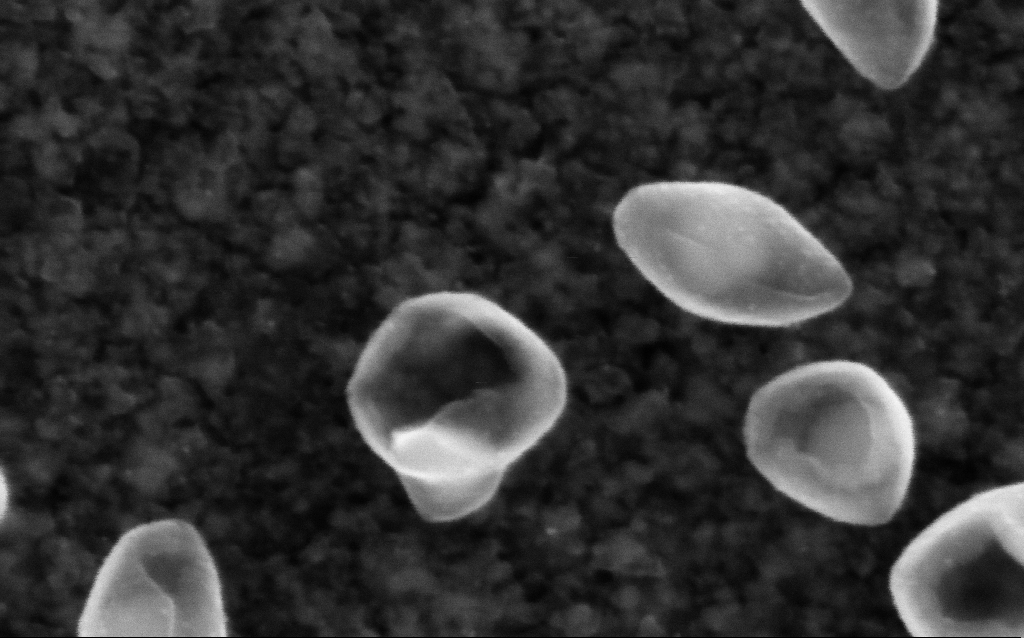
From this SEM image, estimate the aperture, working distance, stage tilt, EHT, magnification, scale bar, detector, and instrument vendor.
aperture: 30 µm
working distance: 2.6 mm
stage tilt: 0°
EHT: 5 kV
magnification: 516.77 K X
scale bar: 100 nm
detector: InLens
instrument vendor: Zeiss